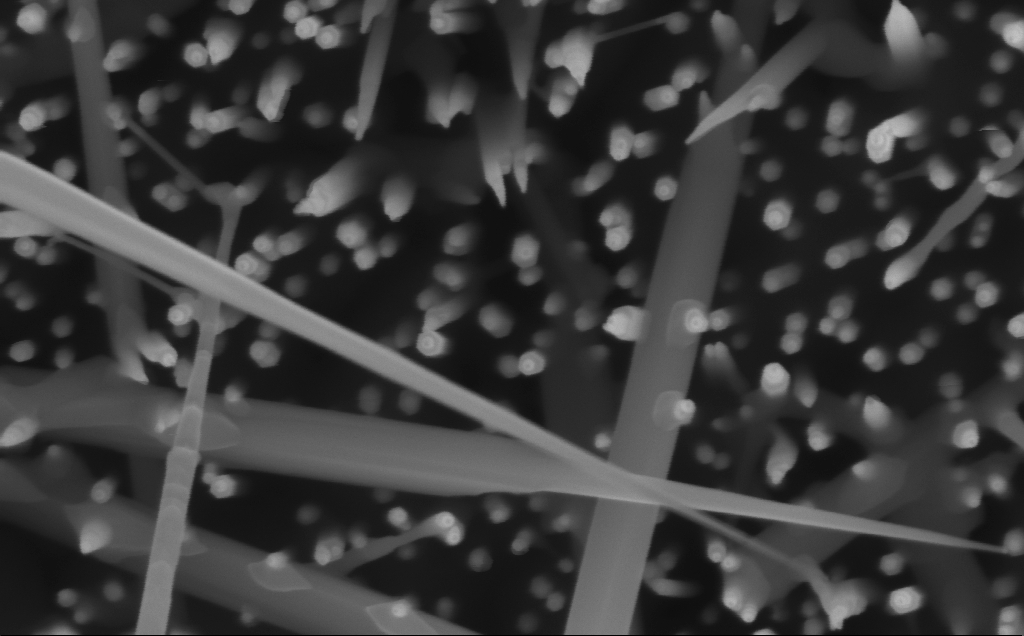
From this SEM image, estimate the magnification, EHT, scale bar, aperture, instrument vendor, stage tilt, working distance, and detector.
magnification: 214.81 K X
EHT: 10 kV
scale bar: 100 nm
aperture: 30 µm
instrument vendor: Zeiss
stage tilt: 0°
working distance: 6 mm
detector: InLens